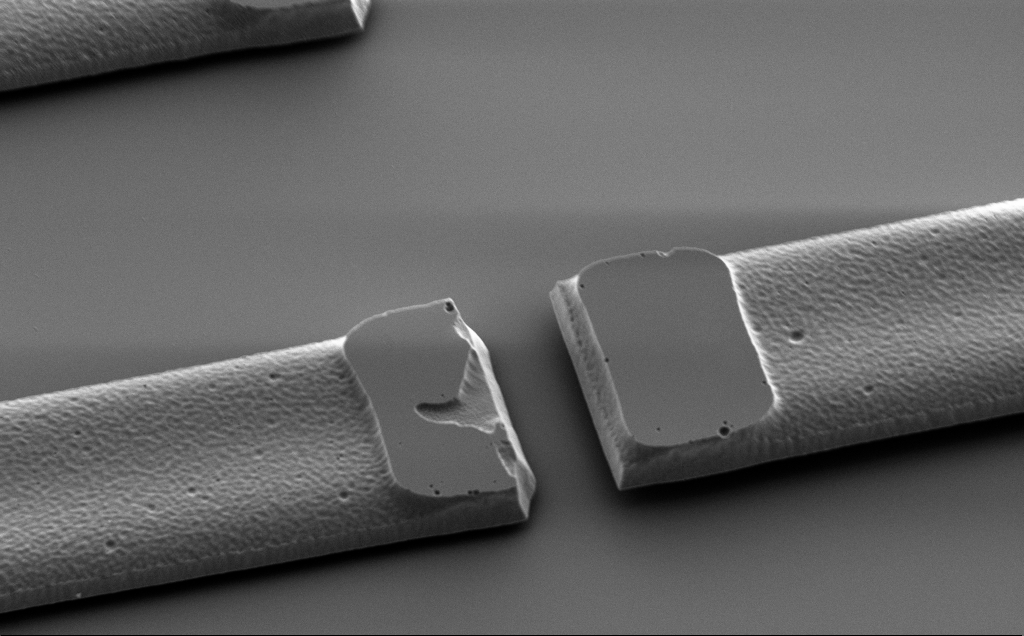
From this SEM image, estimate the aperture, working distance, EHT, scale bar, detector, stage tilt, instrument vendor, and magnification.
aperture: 30 µm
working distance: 10 mm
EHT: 2 kV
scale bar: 10000 nm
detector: SE2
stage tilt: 43°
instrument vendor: Zeiss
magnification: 6.37 K X